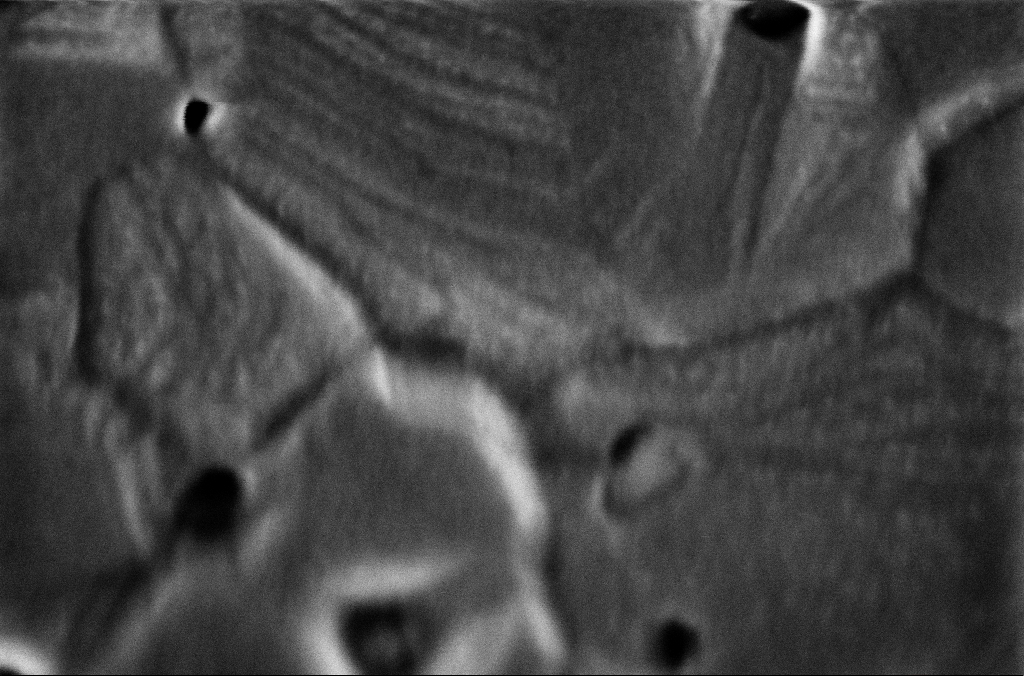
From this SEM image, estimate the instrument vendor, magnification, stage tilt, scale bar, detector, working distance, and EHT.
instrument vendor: Zeiss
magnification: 280 K X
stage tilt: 0°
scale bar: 200 nm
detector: InLens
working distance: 3 mm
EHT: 2 kV